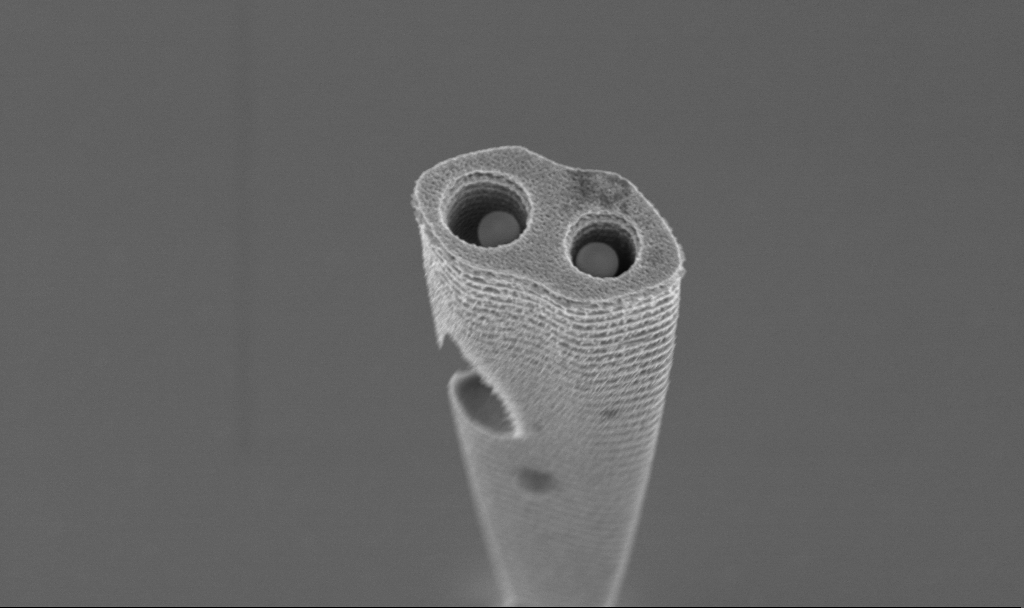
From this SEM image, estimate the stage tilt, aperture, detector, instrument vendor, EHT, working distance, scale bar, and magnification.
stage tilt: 20°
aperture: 30 µm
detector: InLens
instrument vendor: Zeiss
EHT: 3 kV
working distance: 3.1 mm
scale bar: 1000 nm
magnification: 26.67 K X